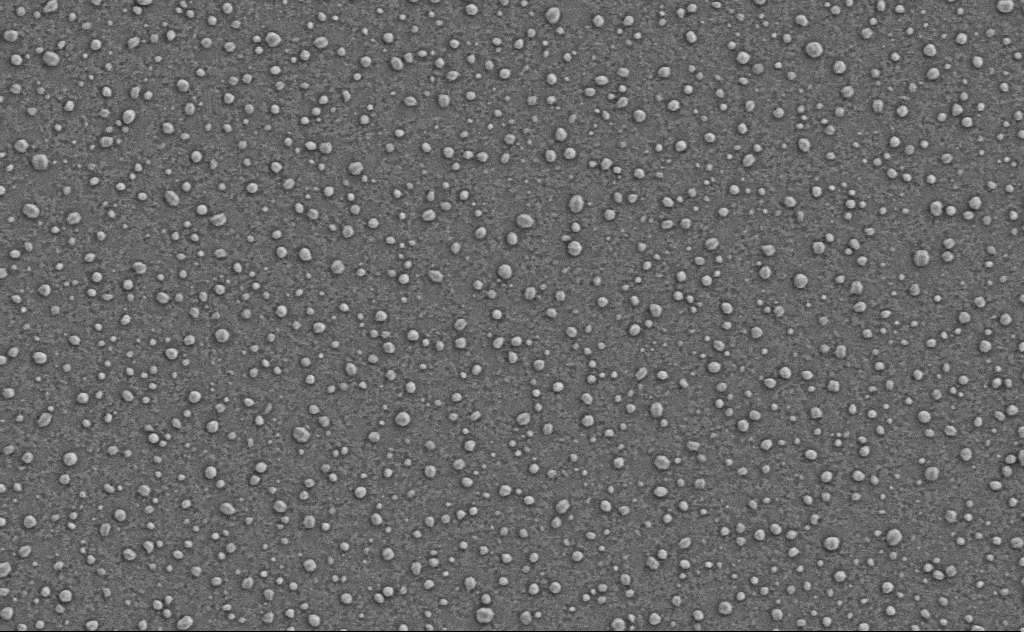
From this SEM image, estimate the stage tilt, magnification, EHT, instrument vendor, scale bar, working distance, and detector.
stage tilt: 0°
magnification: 40 K X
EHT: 3 kV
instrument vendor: Zeiss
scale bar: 1000 nm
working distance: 4 mm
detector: SE2